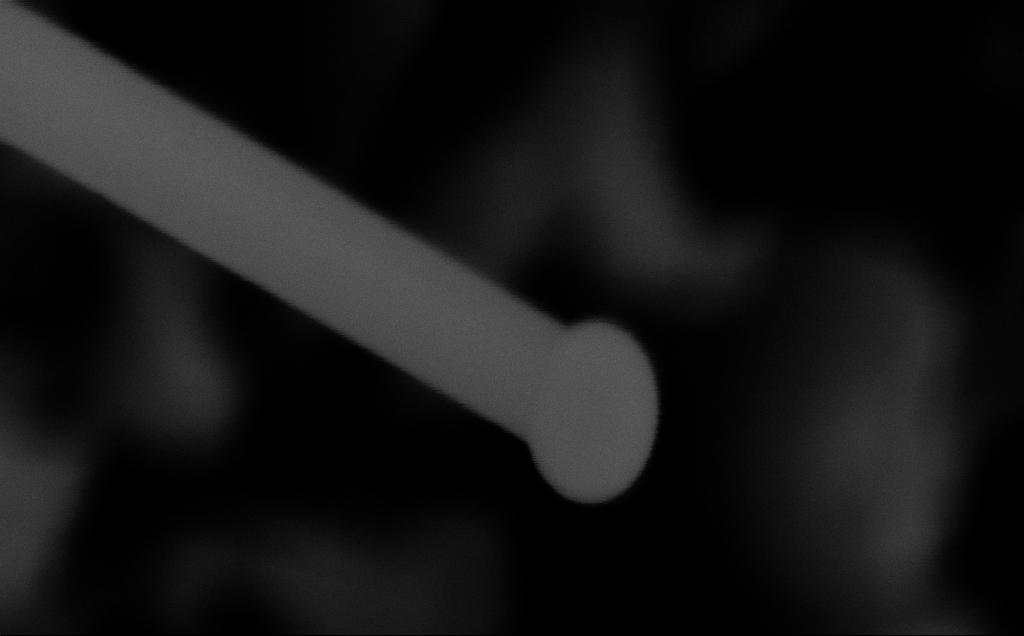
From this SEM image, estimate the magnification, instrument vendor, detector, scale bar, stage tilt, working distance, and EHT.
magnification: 448.37 K X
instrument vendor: Zeiss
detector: InLens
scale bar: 100 nm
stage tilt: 0°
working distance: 6 mm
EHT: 10 kV